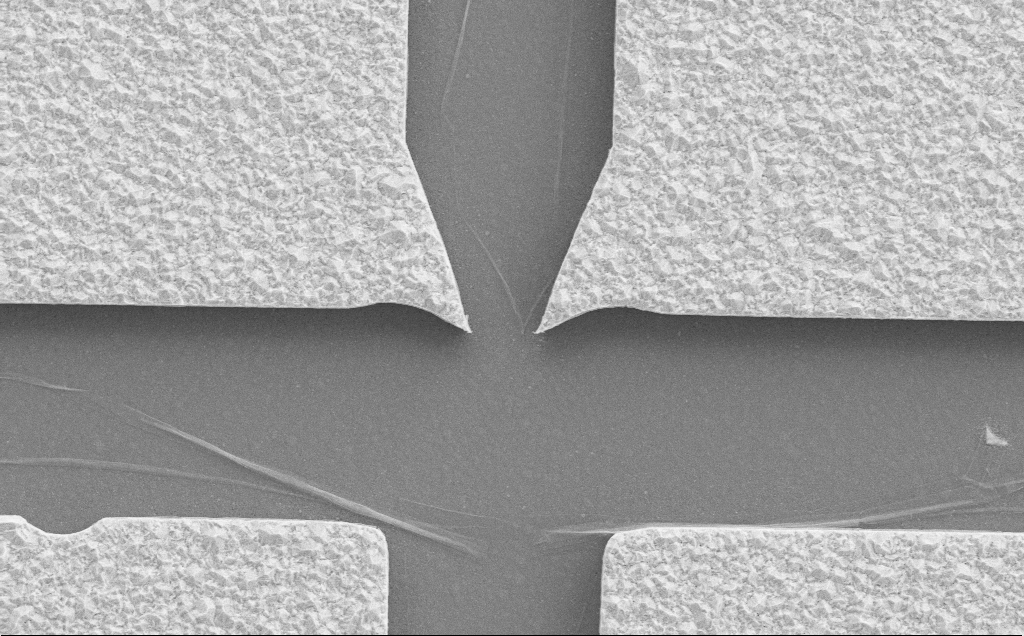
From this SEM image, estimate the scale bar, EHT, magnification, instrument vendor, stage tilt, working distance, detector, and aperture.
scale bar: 2000 nm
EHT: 10 kV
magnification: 7.64 K X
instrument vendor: Zeiss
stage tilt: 0°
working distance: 9 mm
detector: SE2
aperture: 30 µm